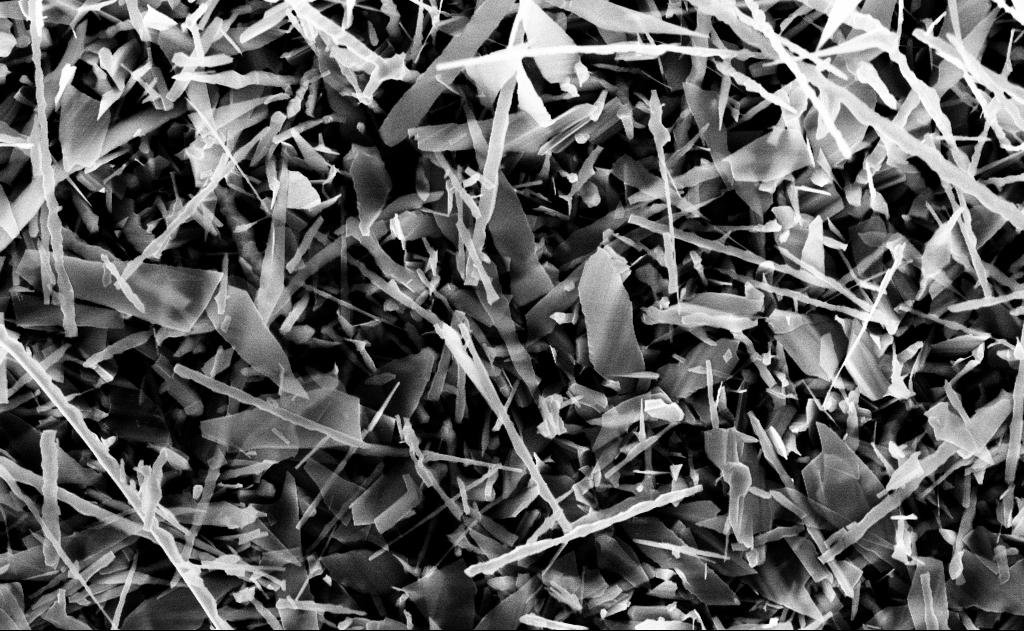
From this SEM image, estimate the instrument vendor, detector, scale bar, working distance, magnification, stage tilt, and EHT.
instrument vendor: Zeiss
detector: InLens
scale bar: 1000 nm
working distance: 13 mm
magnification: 20 K X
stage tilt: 0°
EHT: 10 kV